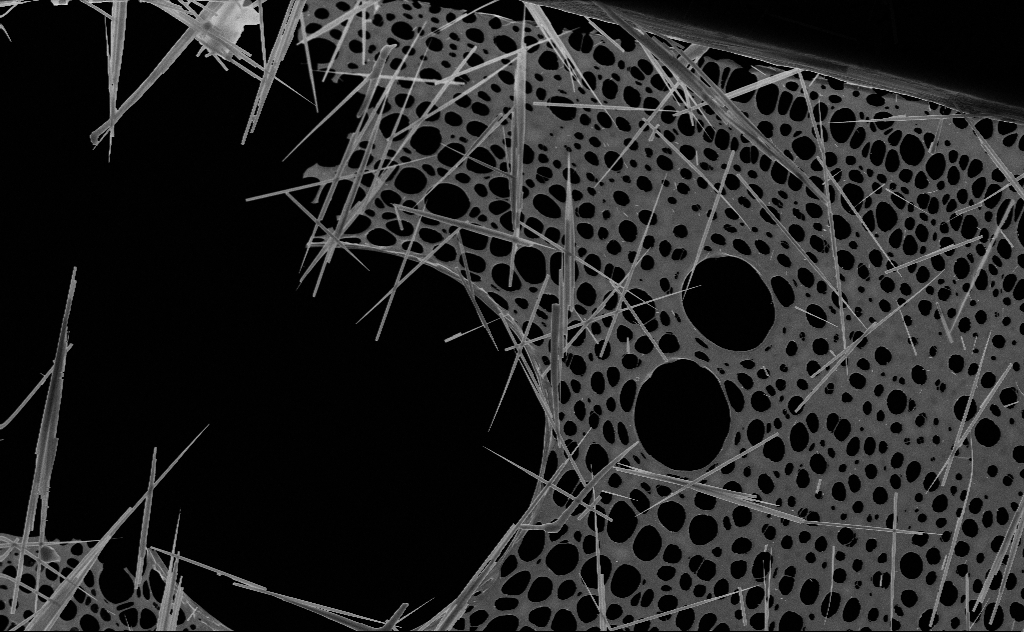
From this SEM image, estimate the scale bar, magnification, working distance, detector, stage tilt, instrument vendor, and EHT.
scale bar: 2000 nm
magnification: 8.51 K X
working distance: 10 mm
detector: SE2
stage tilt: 0°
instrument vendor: Zeiss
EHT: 20 kV